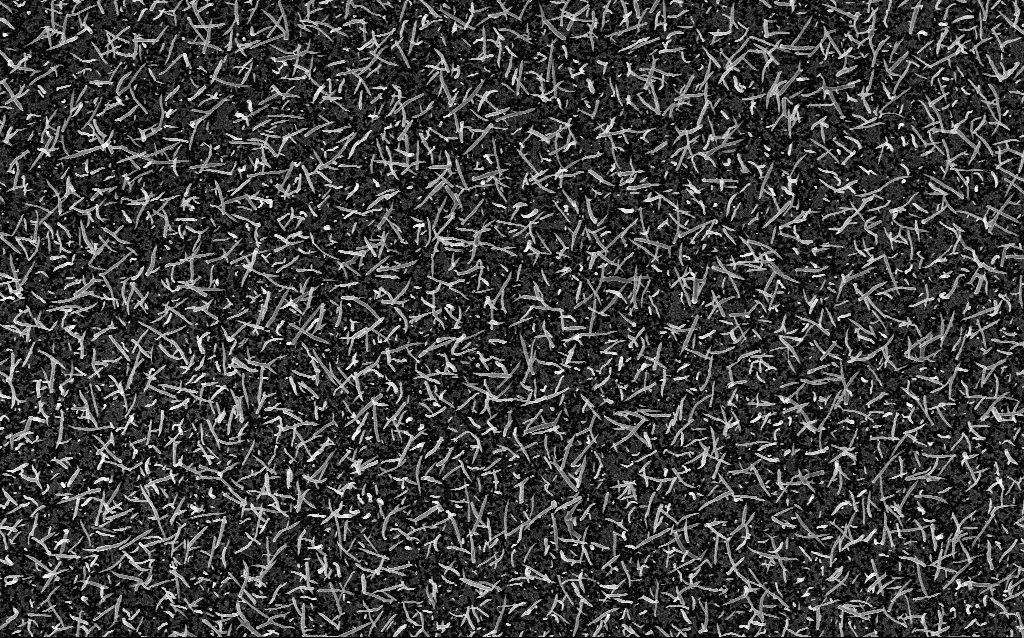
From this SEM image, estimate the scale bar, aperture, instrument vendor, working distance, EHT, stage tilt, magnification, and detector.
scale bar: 2000 nm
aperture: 30 µm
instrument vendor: Zeiss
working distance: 2.6 mm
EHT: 5 kV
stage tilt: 0°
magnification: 10 K X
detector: InLens